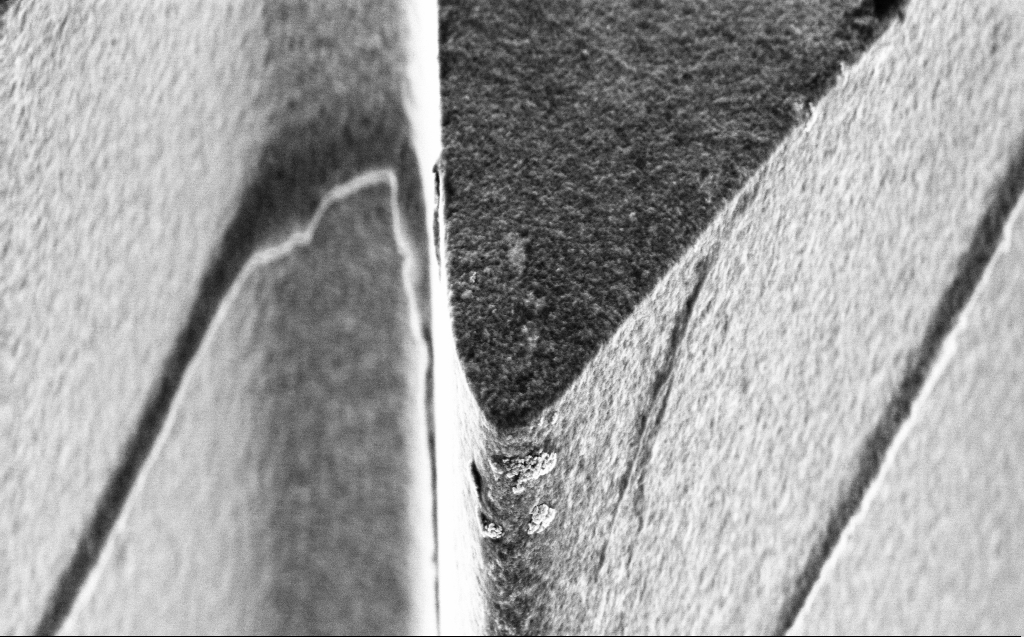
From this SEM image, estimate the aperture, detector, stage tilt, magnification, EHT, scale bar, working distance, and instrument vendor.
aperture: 30 µm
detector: InLens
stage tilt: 45°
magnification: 28.86 K X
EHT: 3 kV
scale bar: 1000 nm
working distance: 4 mm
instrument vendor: Zeiss